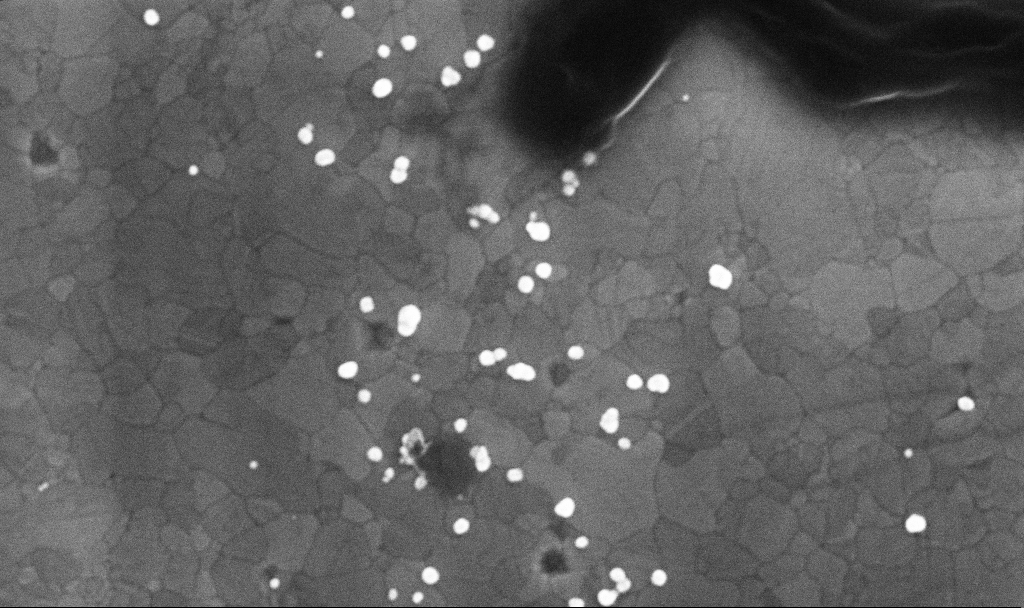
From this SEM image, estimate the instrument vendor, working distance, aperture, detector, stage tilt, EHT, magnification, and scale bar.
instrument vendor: Zeiss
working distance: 3.4 mm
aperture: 30 µm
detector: InLens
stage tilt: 0°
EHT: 10 kV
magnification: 100.45 K X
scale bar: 200 nm